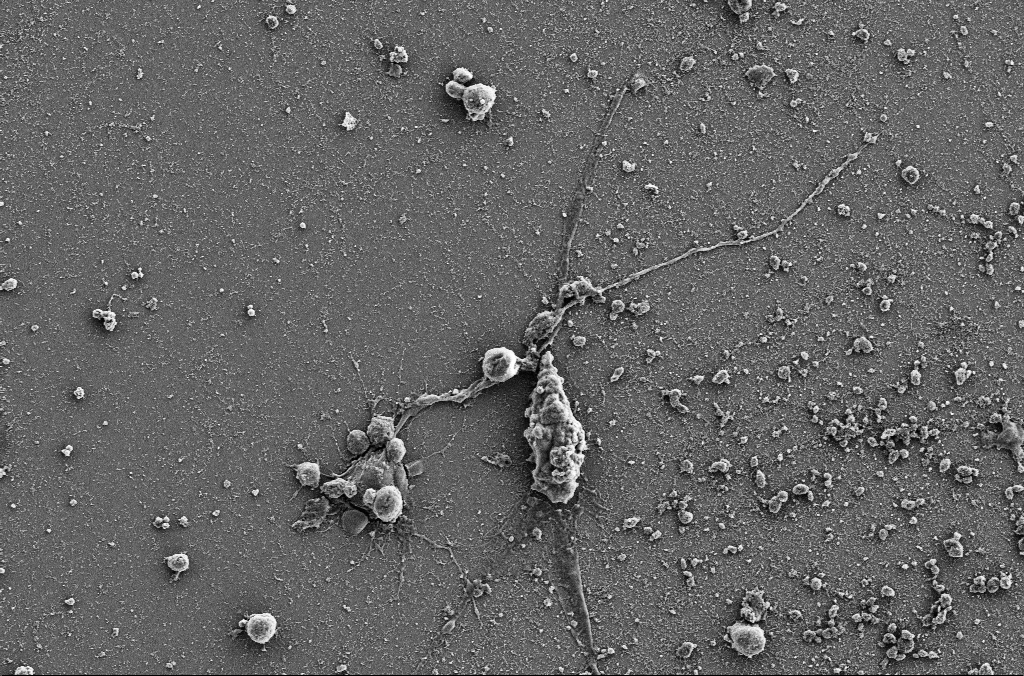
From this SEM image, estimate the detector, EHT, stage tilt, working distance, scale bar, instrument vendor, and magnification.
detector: SE2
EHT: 5 kV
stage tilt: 0°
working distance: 4 mm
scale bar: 20000 nm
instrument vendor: Zeiss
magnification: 2 K X